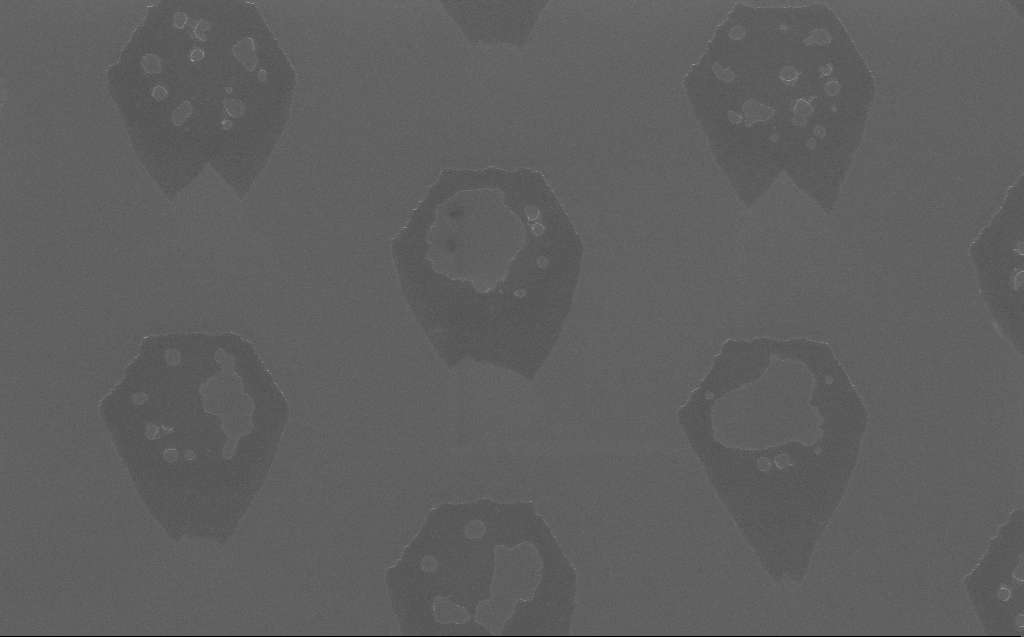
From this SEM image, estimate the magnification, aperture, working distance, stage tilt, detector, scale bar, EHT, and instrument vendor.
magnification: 6.17 K X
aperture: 30 µm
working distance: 6 mm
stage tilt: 0°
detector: InLens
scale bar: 10000 nm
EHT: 5 kV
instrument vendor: Zeiss